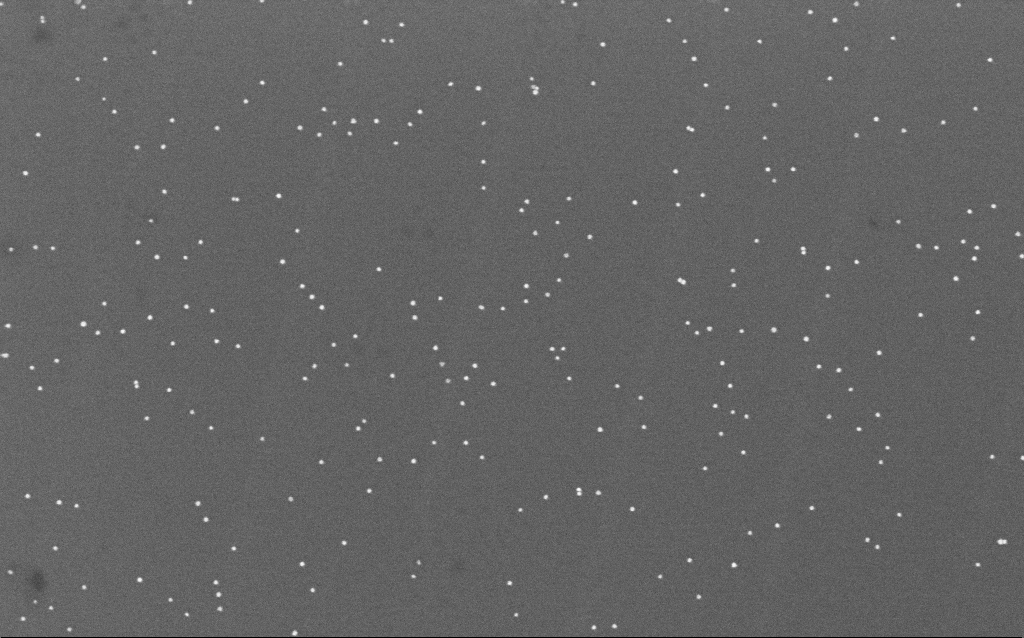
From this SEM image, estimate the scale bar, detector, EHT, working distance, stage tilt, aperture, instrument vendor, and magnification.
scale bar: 200 nm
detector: InLens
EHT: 10 kV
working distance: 6.6 mm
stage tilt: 0°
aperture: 30 µm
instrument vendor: Zeiss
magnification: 100 K X